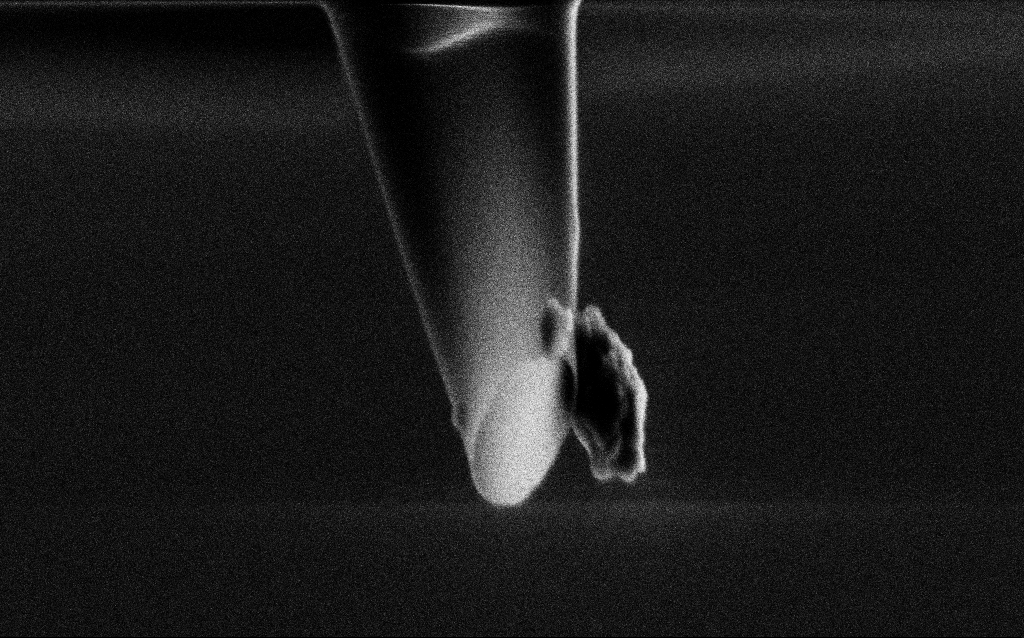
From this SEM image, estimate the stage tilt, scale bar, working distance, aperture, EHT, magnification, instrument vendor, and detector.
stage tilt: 45°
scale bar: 1000 nm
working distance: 5 mm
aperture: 30 µm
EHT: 2 kV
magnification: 65.84 K X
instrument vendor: Zeiss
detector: SE2